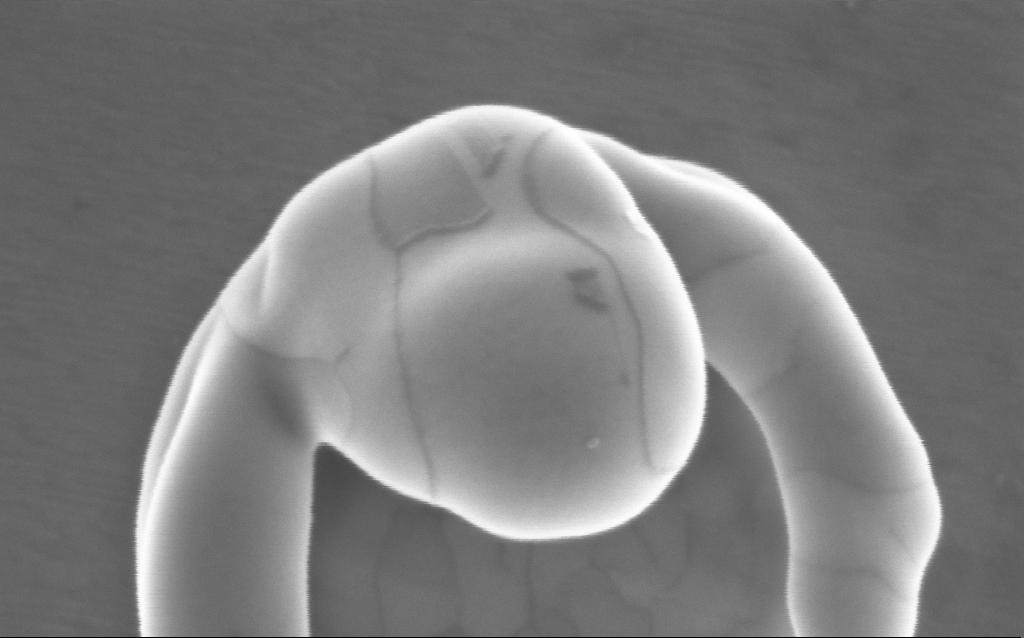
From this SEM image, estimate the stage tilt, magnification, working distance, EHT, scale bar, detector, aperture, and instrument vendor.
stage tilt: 0°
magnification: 274.73 K X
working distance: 4 mm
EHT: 5 kV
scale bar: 100 nm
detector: InLens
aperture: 30 µm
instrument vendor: Zeiss